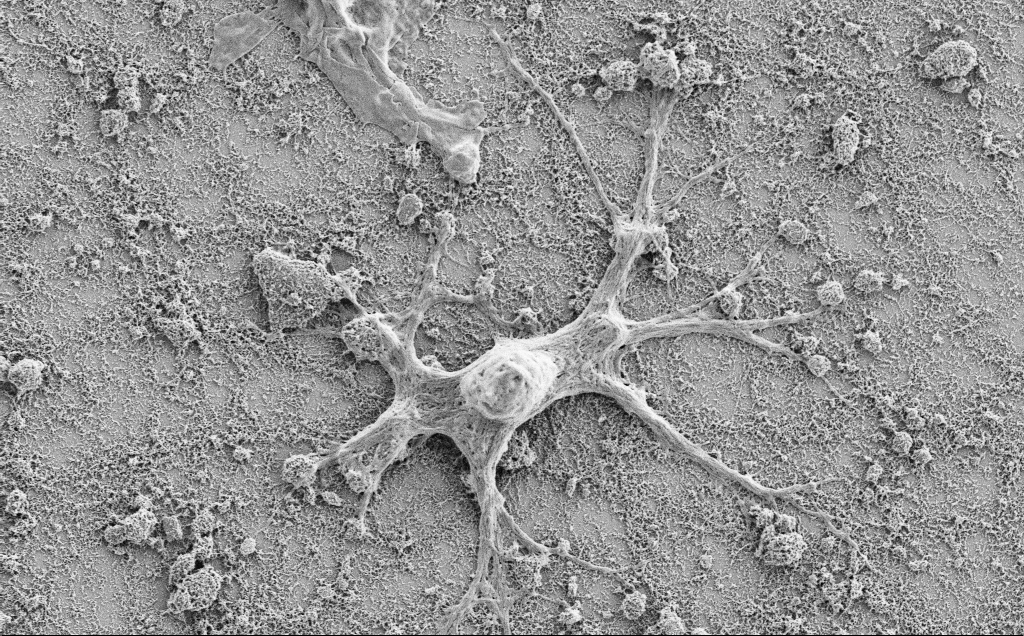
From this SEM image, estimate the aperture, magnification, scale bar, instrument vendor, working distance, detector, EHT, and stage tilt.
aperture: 30 µm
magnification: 5 K X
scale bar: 10000 nm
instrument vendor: Zeiss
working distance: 7.1 mm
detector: SE2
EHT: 2 kV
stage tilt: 0°